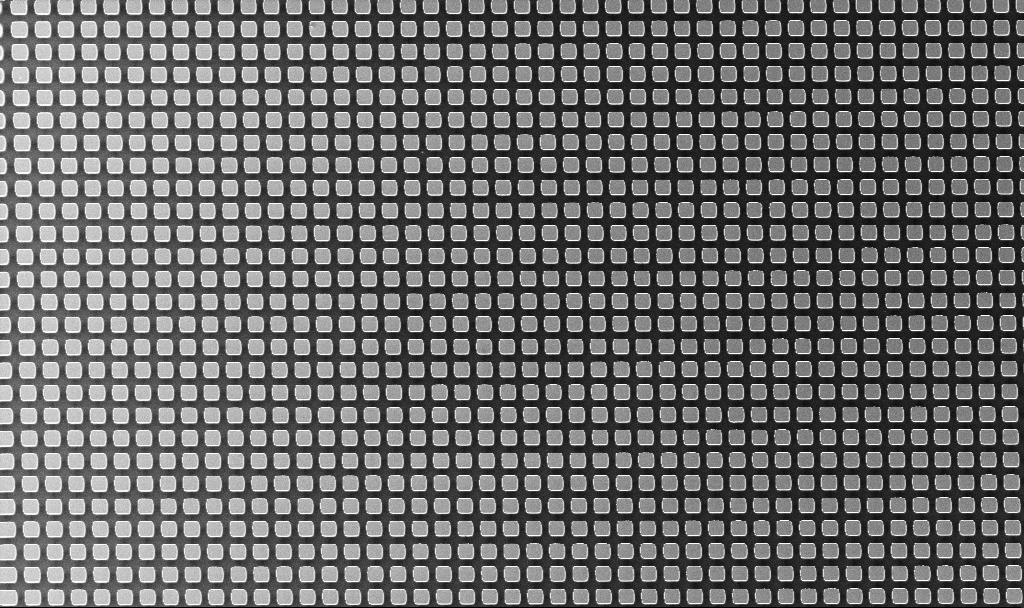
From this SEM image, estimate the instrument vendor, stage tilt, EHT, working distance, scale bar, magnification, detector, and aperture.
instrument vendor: Zeiss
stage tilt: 0°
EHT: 3 kV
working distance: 3.2 mm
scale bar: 10000 nm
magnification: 1.41 K X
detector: InLens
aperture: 30 µm